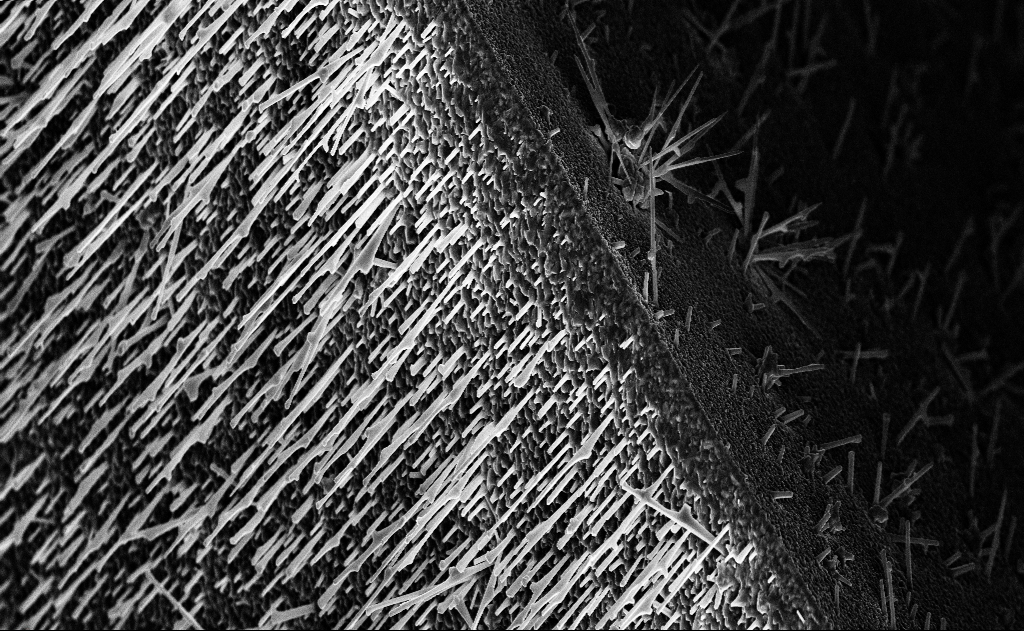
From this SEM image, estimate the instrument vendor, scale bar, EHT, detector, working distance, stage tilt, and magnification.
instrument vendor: Zeiss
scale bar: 10000 nm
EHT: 10 kV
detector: InLens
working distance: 15 mm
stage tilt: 0°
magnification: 5 K X